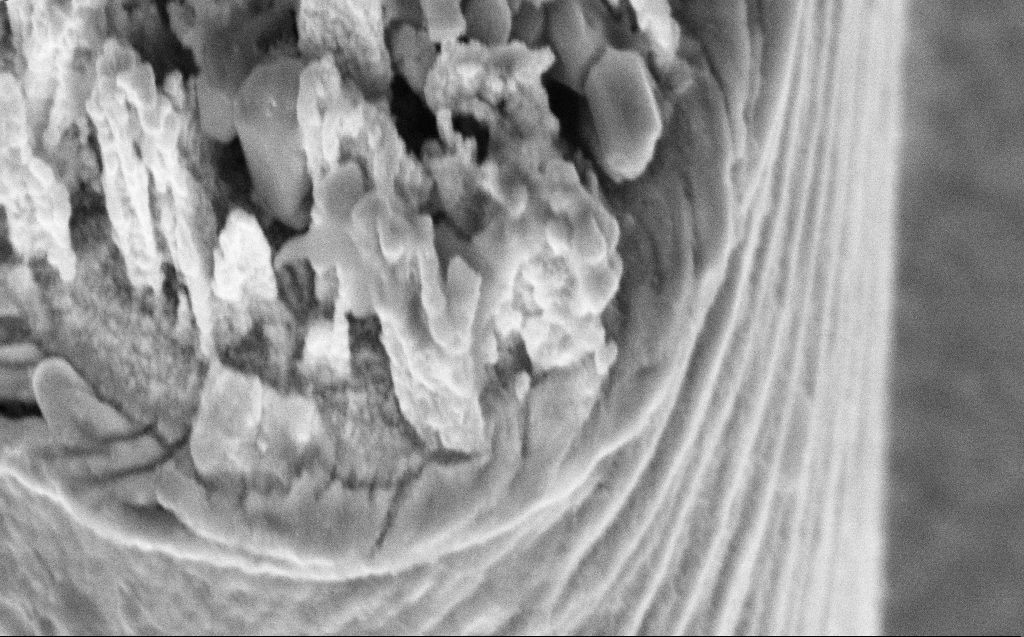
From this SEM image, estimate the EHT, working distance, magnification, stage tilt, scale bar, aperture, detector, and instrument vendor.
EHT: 5 kV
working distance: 9 mm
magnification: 92.98 K X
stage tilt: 45°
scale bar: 200 nm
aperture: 30 µm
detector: InLens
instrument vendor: Zeiss